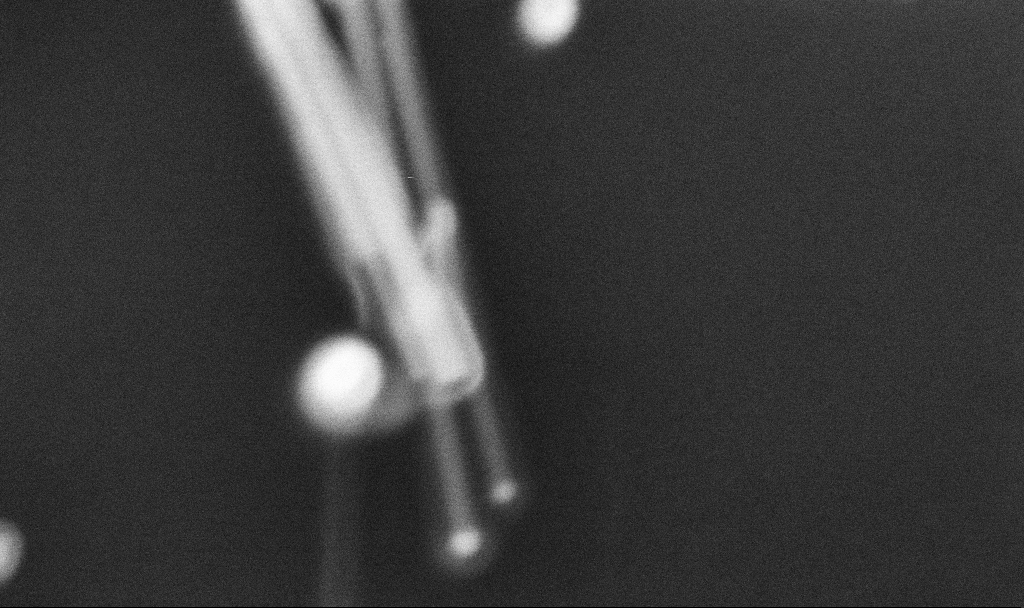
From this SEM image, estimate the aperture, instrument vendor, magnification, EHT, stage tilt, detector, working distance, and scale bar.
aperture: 30 µm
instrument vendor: Zeiss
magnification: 324.03 K X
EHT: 5 kV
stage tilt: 0°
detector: InLens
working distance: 3.3 mm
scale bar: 200 nm